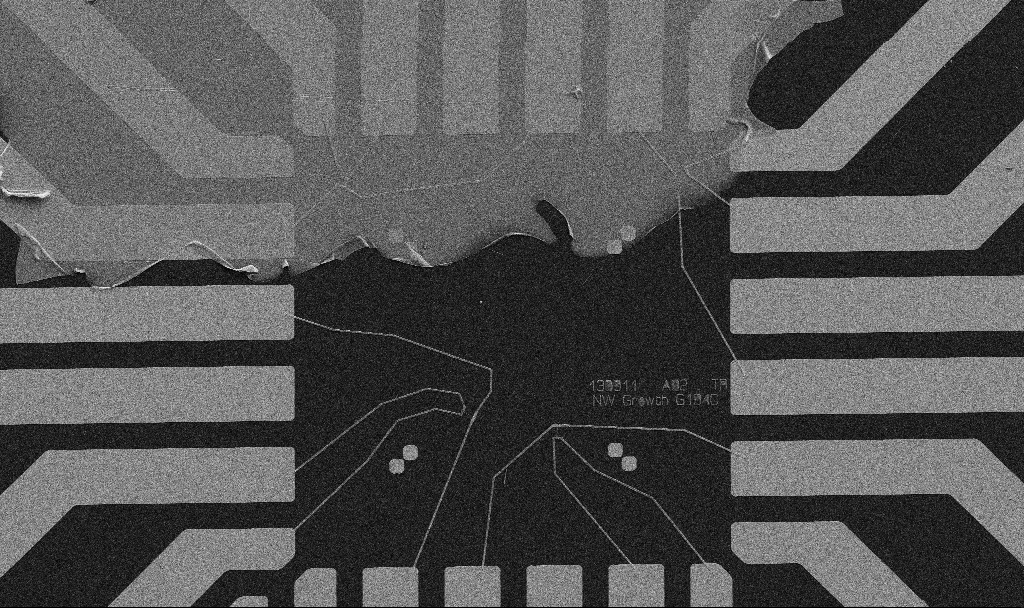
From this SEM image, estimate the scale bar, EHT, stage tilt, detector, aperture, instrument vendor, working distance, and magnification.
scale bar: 20000 nm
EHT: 5 kV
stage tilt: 0°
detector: SE2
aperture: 30 µm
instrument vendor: Zeiss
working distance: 10.7 mm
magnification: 1 K X